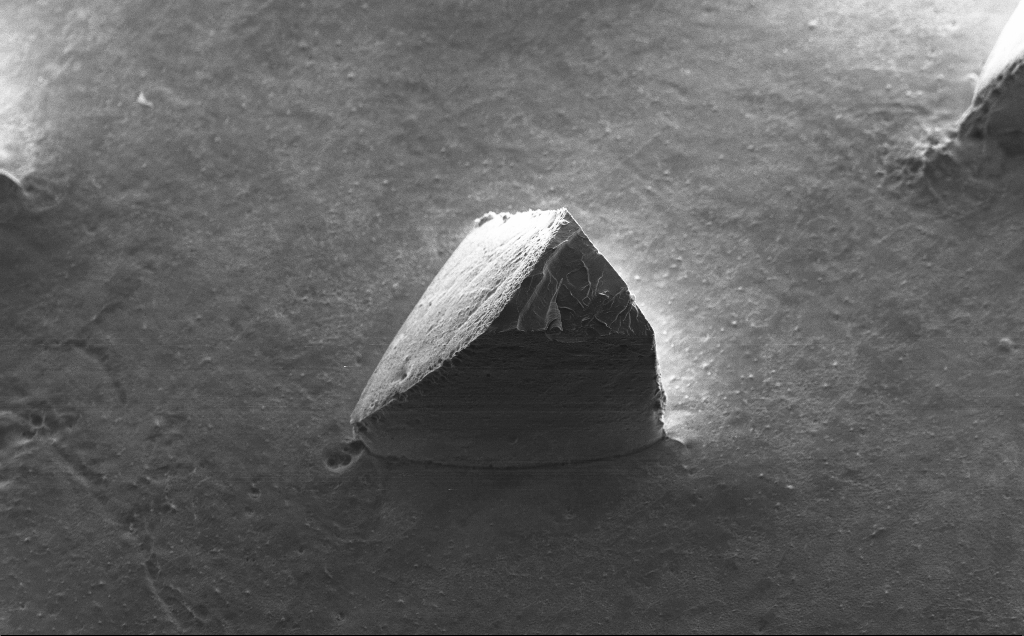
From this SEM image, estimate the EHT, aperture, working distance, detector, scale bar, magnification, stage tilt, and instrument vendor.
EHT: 10 kV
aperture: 30 µm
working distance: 9 mm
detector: SE2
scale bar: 200000 nm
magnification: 0.195 K X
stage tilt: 30°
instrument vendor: Zeiss